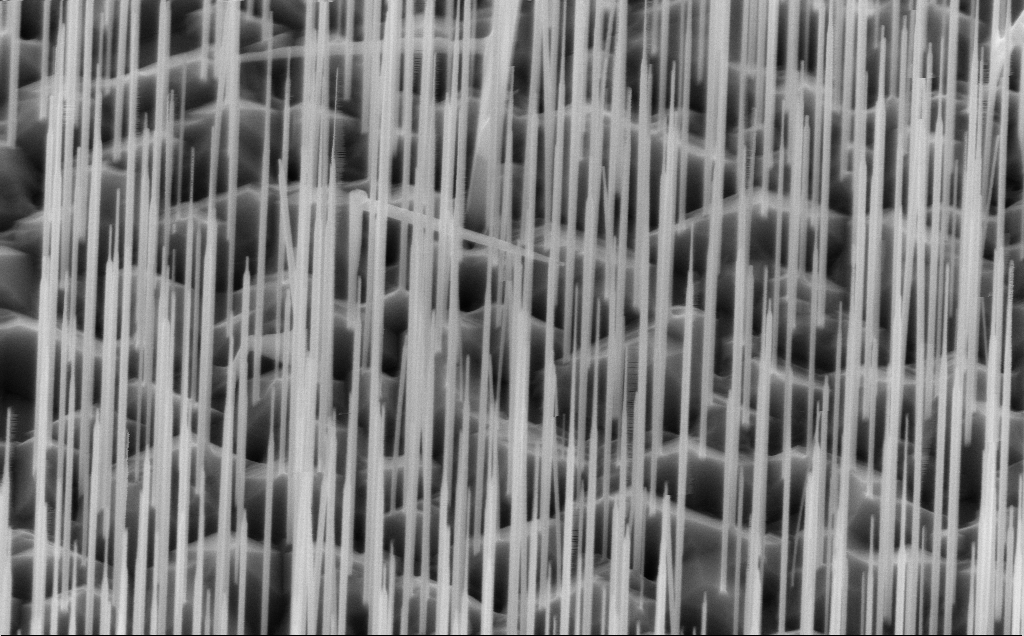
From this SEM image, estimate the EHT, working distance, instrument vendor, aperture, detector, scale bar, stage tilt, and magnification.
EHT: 10 kV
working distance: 5 mm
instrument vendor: Zeiss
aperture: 30 µm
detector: InLens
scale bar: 1000 nm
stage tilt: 45°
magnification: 40 K X